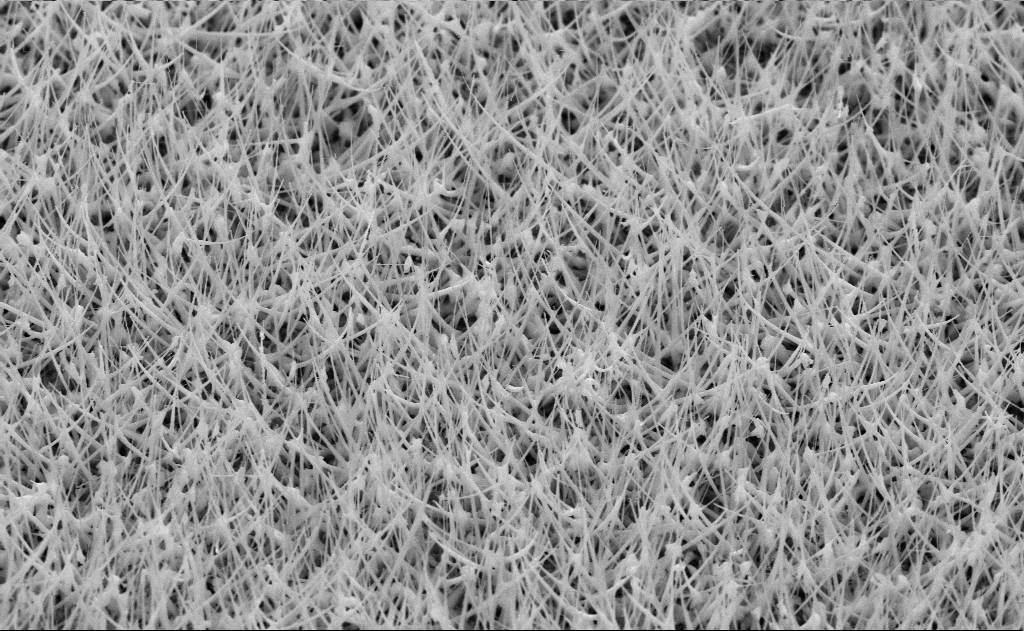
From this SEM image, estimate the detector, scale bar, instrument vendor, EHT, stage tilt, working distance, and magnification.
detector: SE2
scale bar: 2000 nm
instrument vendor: Zeiss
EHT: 10 kV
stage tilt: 45°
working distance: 11 mm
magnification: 20 K X